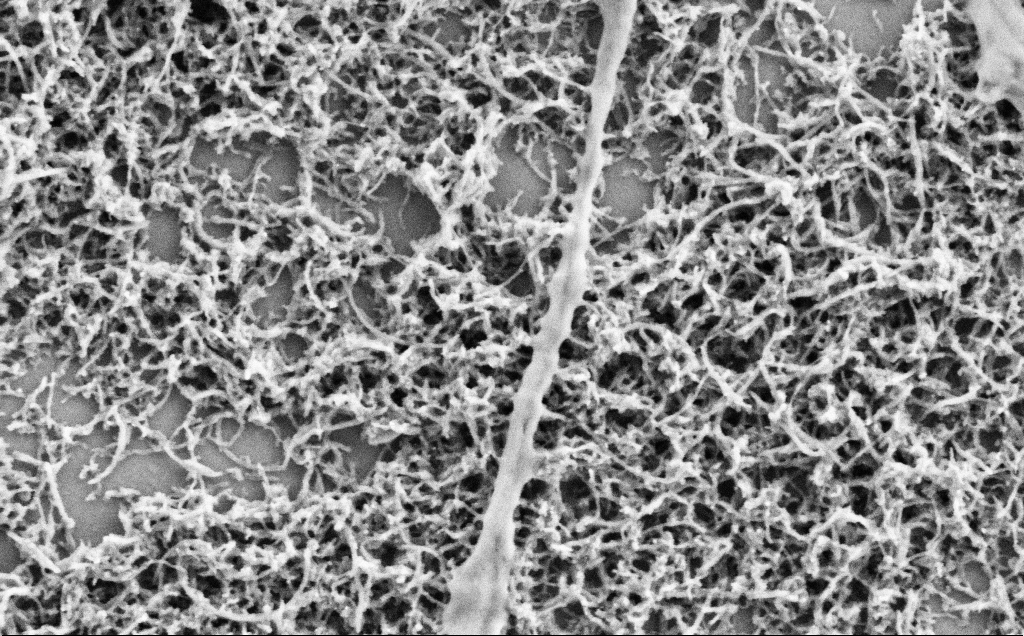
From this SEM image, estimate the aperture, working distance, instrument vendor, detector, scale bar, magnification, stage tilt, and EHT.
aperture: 30 µm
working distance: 7.1 mm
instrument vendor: Zeiss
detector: SE2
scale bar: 1000 nm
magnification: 50 K X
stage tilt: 0°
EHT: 2 kV